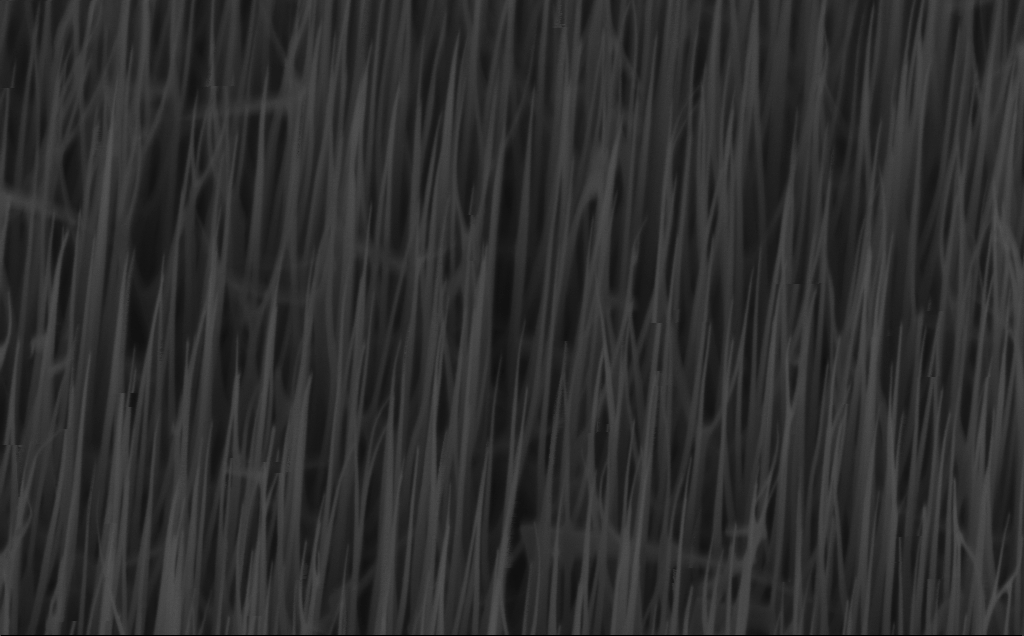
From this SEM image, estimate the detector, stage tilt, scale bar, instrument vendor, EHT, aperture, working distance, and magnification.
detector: InLens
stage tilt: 45°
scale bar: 1000 nm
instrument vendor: Zeiss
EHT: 3 kV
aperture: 30 µm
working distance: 6 mm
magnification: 40 K X